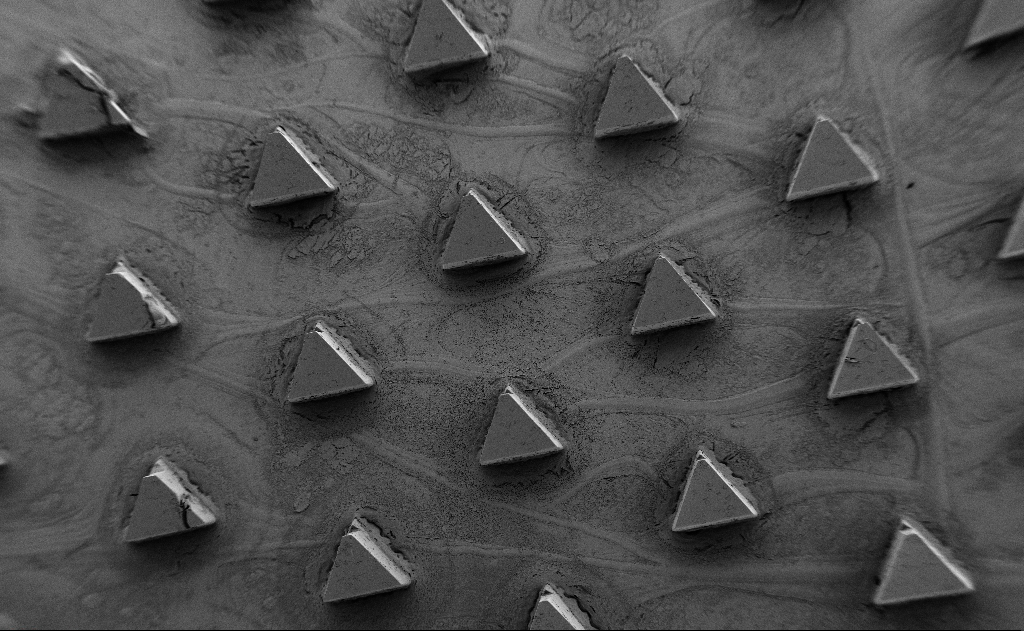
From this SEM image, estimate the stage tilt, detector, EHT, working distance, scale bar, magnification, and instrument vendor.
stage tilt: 0°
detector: SE2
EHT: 5 kV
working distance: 13 mm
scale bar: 200000 nm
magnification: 0.075 K X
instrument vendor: Zeiss